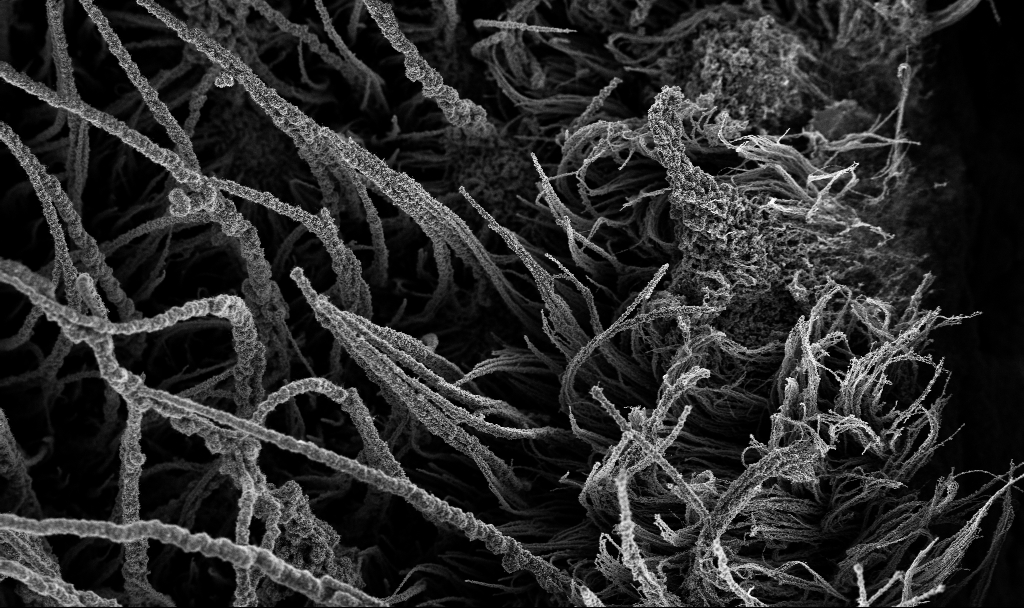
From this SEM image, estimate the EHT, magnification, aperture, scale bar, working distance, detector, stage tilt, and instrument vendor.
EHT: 3 kV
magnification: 0.25 K X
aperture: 30 µm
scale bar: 100000 nm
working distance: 3.4 mm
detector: InLens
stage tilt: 0°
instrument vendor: Zeiss